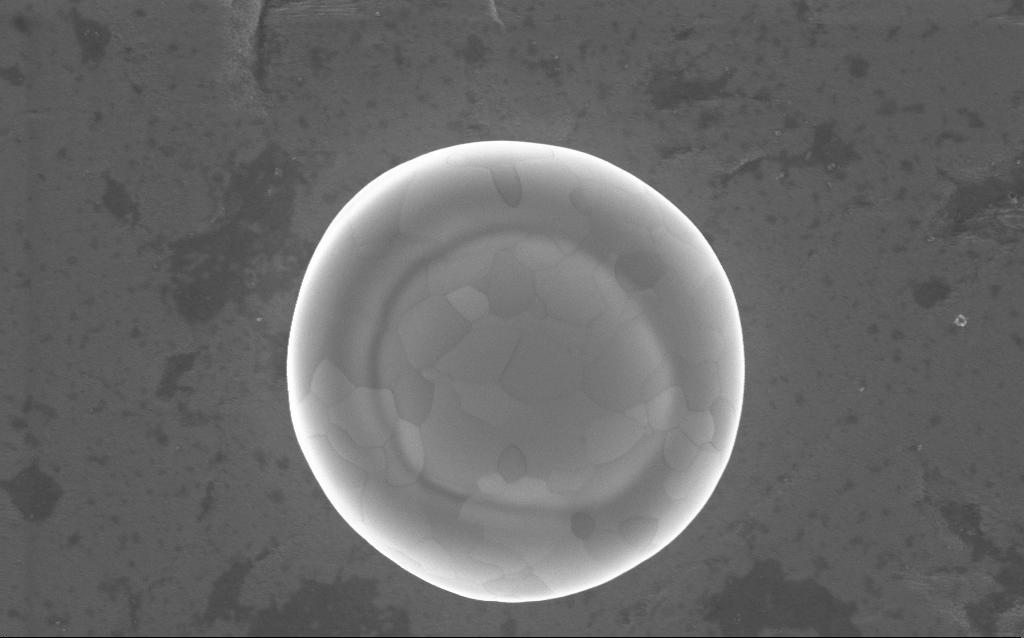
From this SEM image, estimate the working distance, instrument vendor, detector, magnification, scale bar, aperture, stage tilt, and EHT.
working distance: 4 mm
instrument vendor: Zeiss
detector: InLens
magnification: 40 K X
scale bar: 1000 nm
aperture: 30 µm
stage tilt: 0°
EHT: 5 kV